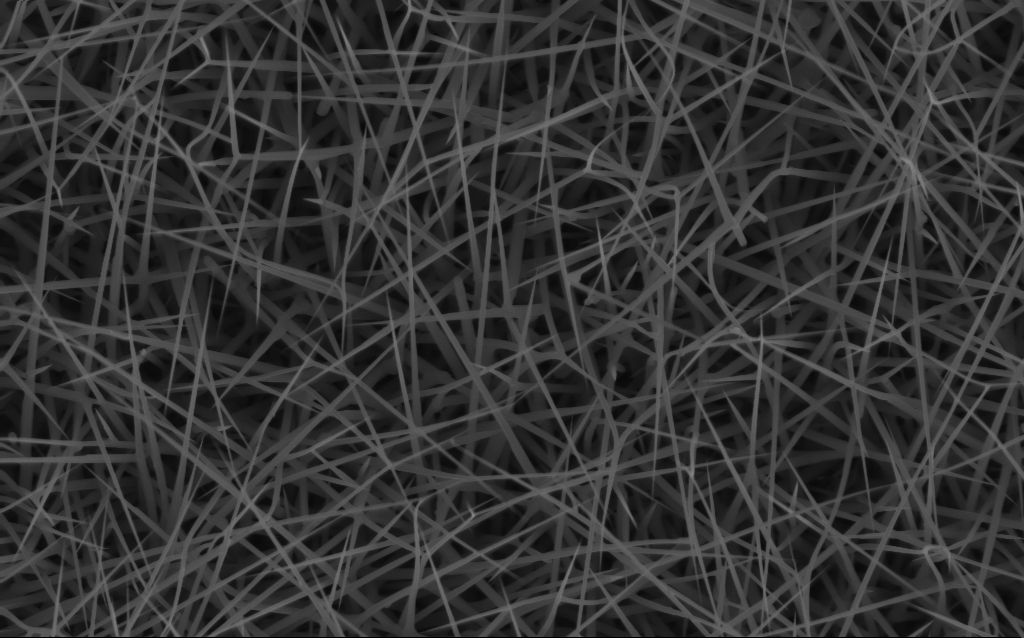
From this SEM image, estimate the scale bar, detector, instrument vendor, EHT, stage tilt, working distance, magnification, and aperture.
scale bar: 2000 nm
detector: InLens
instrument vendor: Zeiss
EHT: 10 kV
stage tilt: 0°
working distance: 6 mm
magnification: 20 K X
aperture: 30 µm